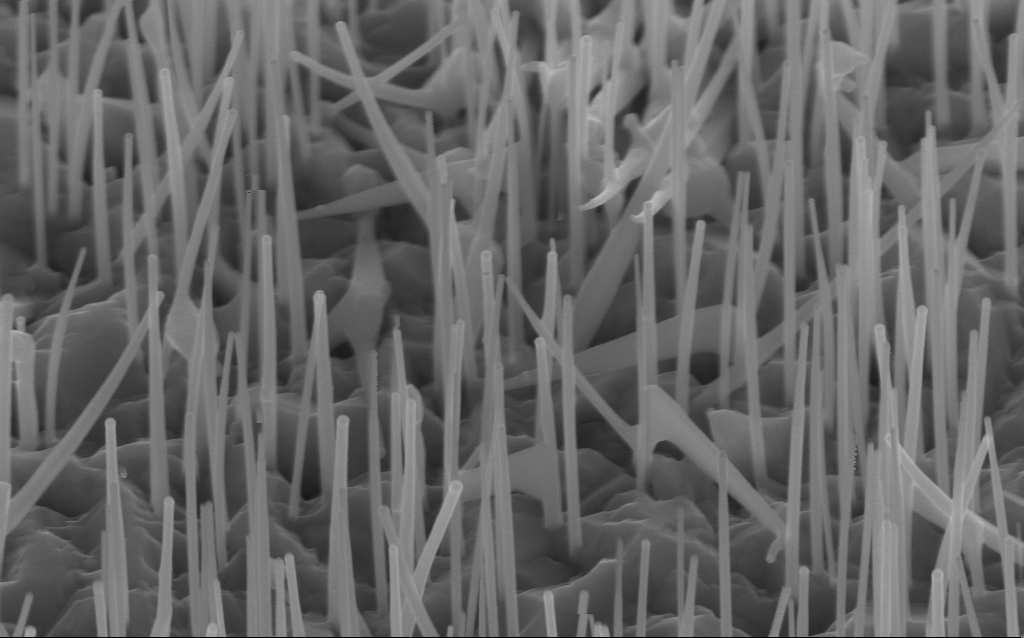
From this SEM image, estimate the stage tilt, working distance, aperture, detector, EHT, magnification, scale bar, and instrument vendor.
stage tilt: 45°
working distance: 6 mm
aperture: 30 µm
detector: InLens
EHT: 10 kV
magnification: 75.68 K X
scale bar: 200 nm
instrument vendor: Zeiss